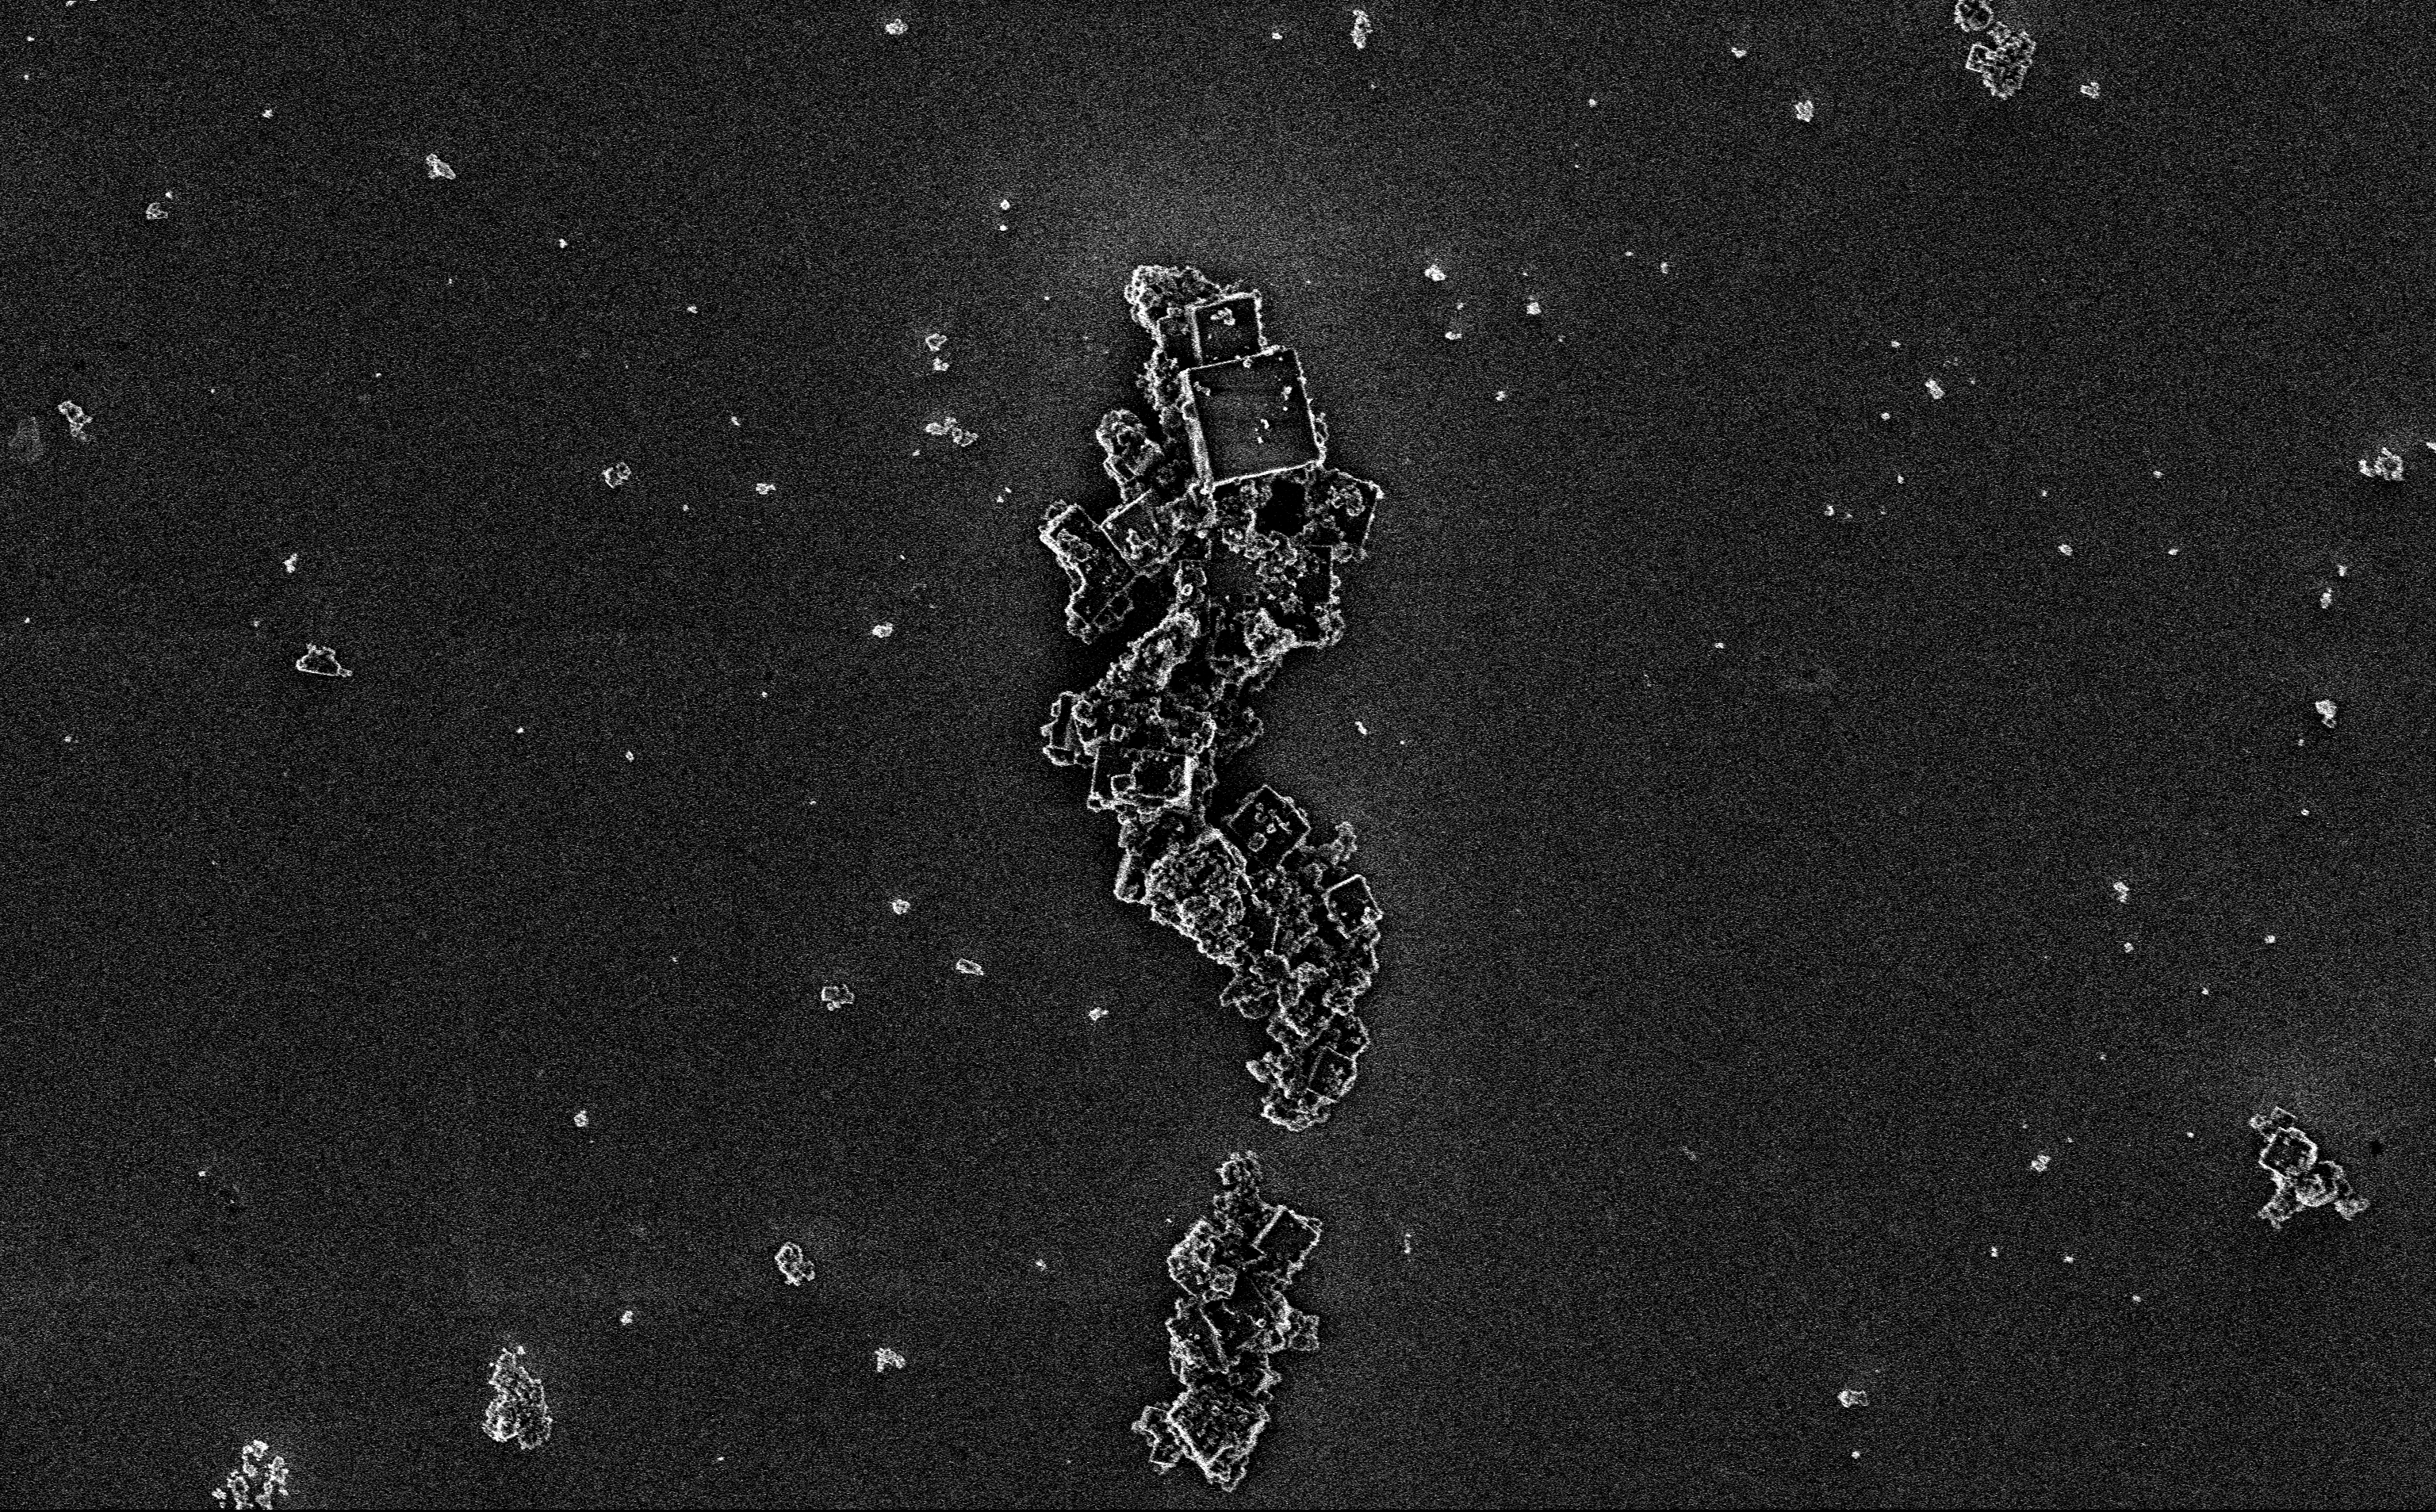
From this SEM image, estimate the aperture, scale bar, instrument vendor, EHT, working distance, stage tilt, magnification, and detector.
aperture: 30 µm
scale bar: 10000 nm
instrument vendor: Zeiss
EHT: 3 kV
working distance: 3 mm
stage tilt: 0°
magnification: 4.69 K X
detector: InLens